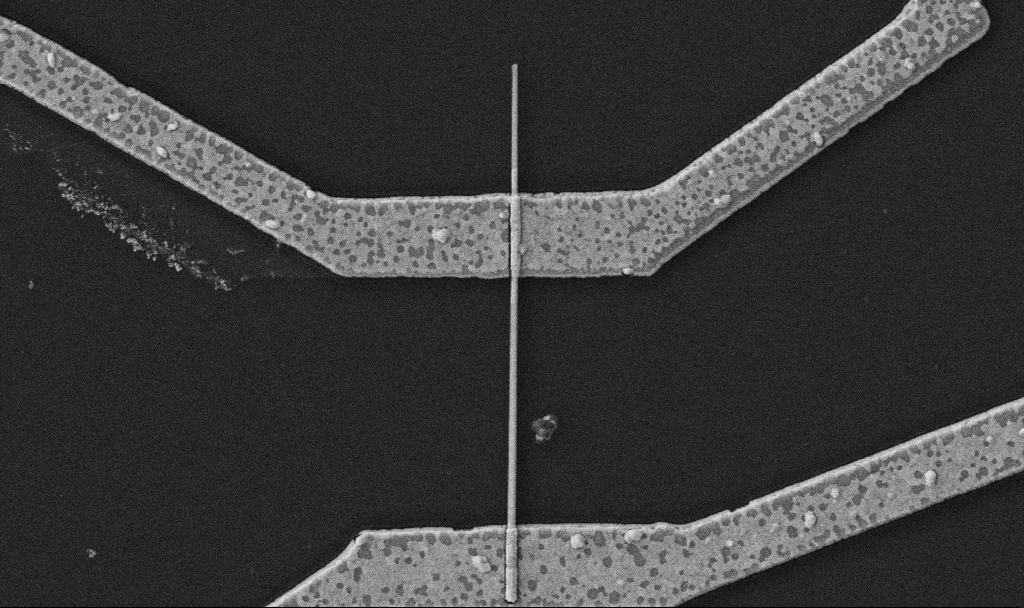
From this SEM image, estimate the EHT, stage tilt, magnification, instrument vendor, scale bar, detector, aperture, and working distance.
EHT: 5 kV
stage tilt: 0°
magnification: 30 K X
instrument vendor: Zeiss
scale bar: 1000 nm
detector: SE2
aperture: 30 µm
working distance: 10.7 mm